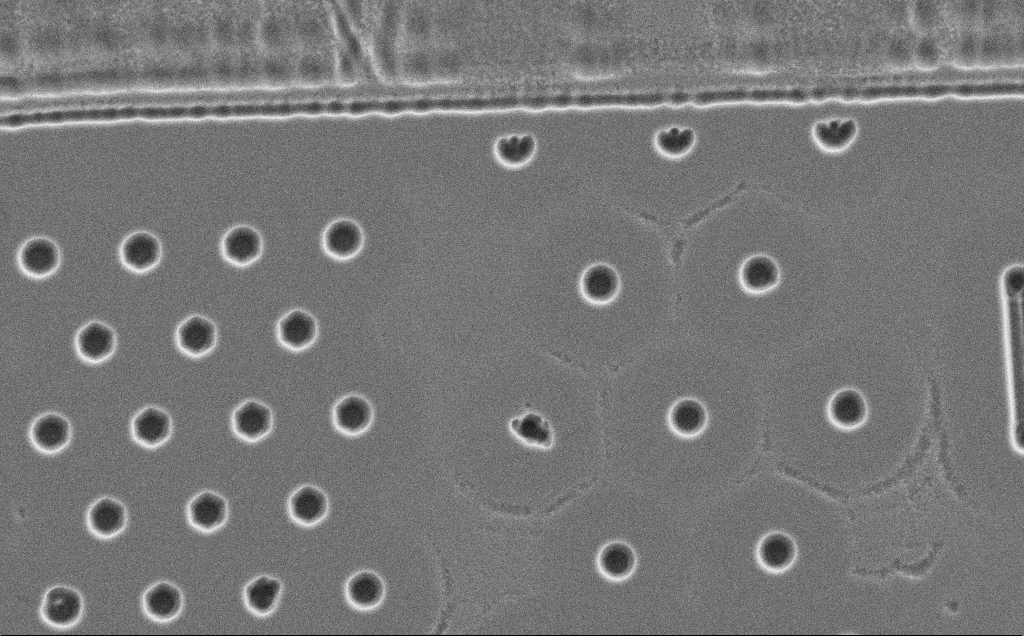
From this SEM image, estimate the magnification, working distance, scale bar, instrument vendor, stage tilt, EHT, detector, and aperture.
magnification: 5.81 K X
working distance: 13 mm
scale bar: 10000 nm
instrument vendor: Zeiss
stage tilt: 0°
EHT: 5 kV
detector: SE2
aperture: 30 µm